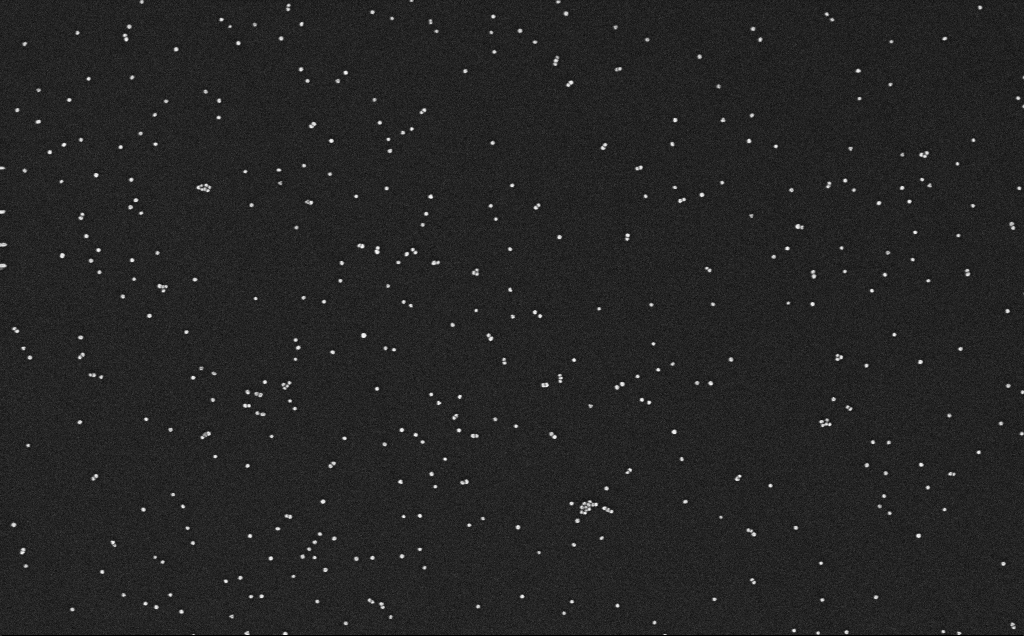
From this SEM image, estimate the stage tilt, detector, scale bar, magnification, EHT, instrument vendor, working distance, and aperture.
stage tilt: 0°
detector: InLens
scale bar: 200 nm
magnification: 100 K X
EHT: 10 kV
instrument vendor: Zeiss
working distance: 3.2 mm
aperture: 30 µm